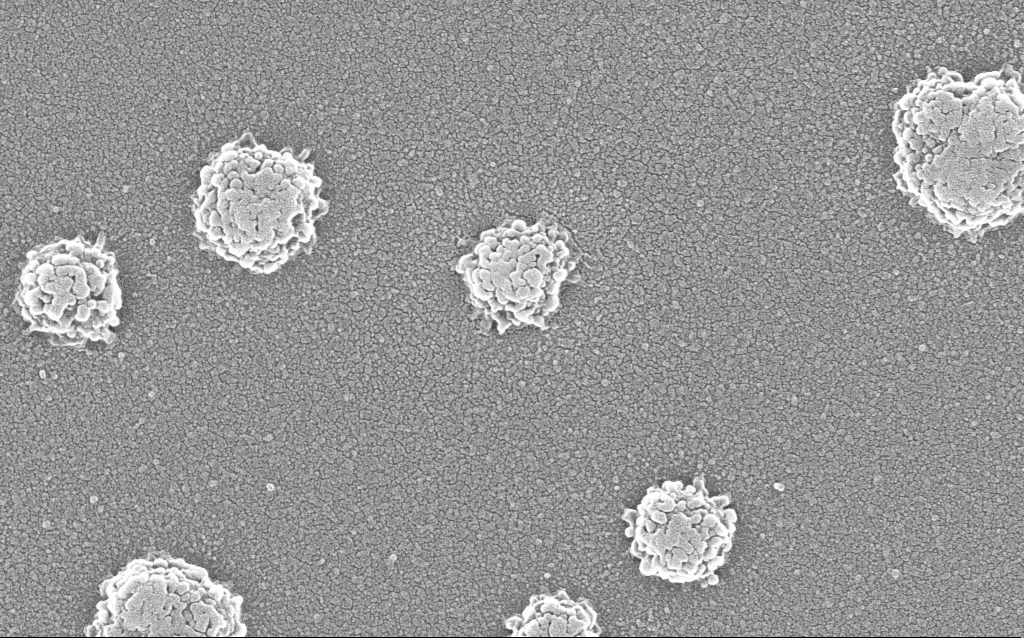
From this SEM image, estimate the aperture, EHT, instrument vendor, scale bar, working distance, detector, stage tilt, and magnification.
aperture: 30 µm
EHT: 20 kV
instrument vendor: Zeiss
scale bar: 200 nm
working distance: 1.8 mm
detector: InLens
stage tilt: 0°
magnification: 100 K X